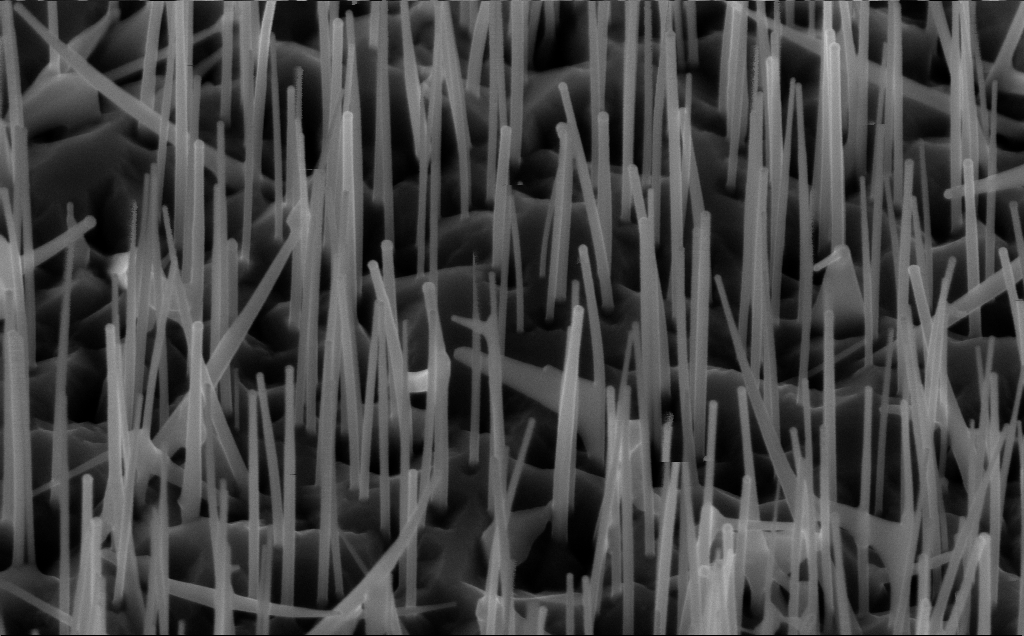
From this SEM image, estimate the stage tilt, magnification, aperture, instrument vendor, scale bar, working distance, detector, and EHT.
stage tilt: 45°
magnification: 80 K X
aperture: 30 µm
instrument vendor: Zeiss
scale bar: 200 nm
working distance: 5 mm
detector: InLens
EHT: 10 kV